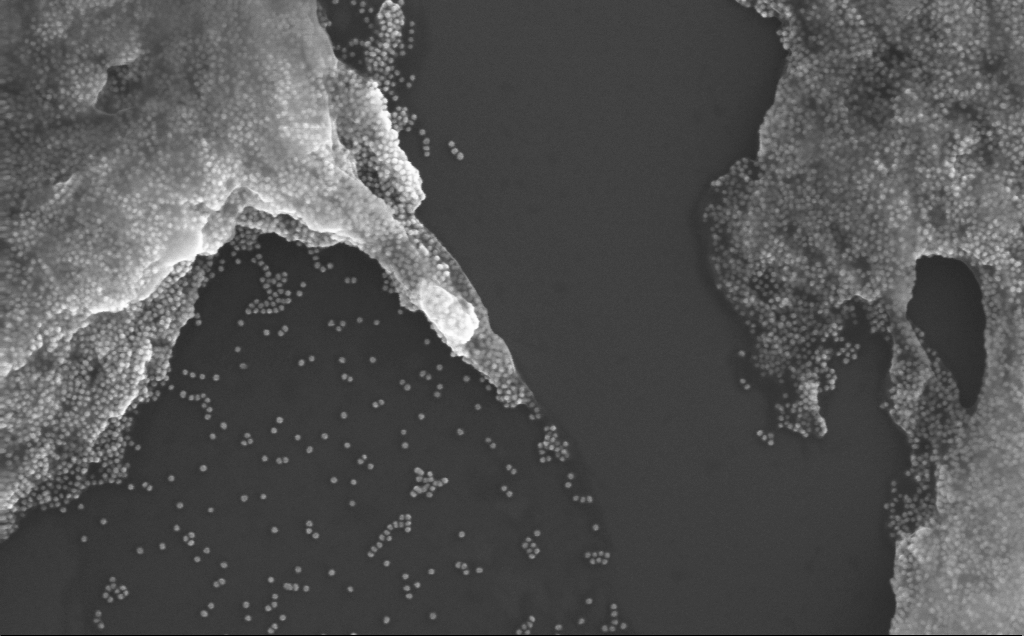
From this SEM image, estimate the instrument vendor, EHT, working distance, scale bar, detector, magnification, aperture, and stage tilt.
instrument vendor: Zeiss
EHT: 10 kV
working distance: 3 mm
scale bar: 200 nm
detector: InLens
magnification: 130.6 K X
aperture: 30 µm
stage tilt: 0°